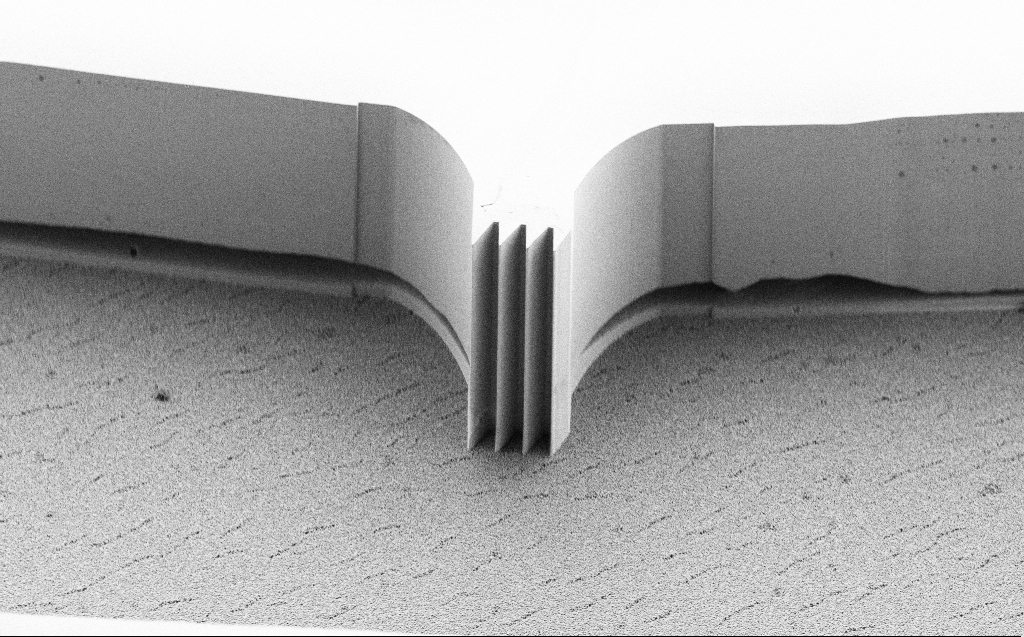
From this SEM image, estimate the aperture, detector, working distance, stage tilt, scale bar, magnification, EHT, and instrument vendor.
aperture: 30 µm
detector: SE2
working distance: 5 mm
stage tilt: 45°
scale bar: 20000 nm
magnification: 0.897 K X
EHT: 5 kV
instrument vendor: Zeiss